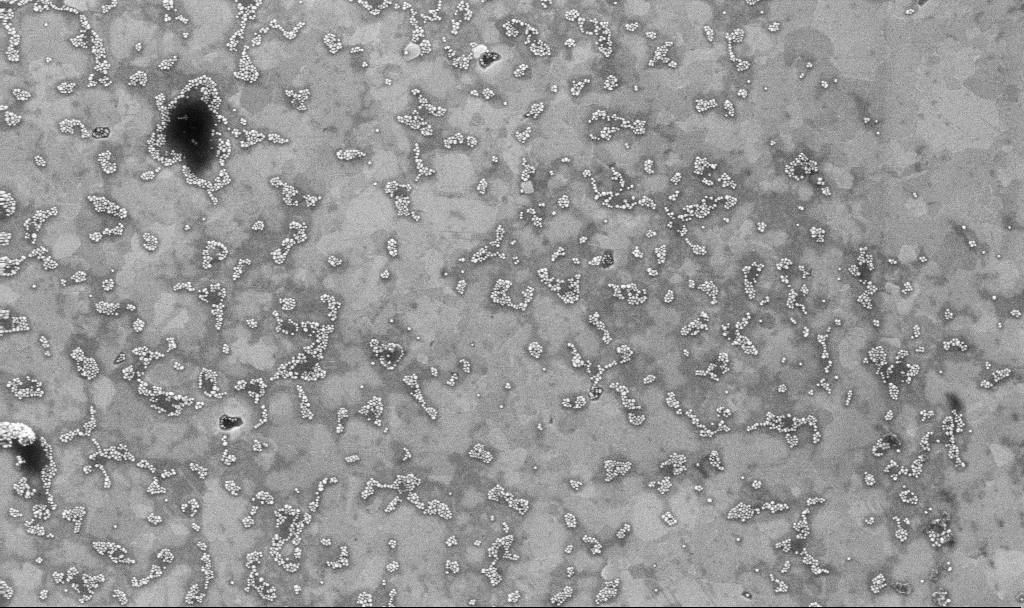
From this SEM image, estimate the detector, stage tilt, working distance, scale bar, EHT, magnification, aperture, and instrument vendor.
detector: InLens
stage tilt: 0°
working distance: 3.1 mm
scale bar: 1000 nm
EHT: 10 kV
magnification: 50.18 K X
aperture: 30 µm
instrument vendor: Zeiss